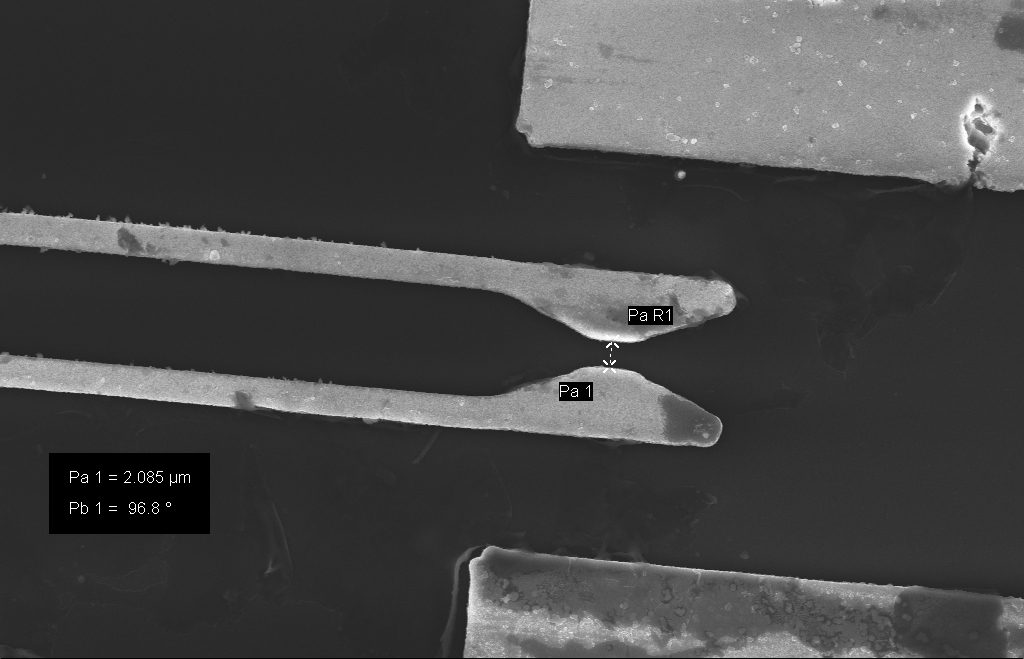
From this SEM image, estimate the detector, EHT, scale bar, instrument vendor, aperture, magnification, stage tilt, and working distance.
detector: InLens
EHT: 10 kV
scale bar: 10000 nm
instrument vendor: Zeiss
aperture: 30 µm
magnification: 4.43 K X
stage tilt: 0°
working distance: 7 mm